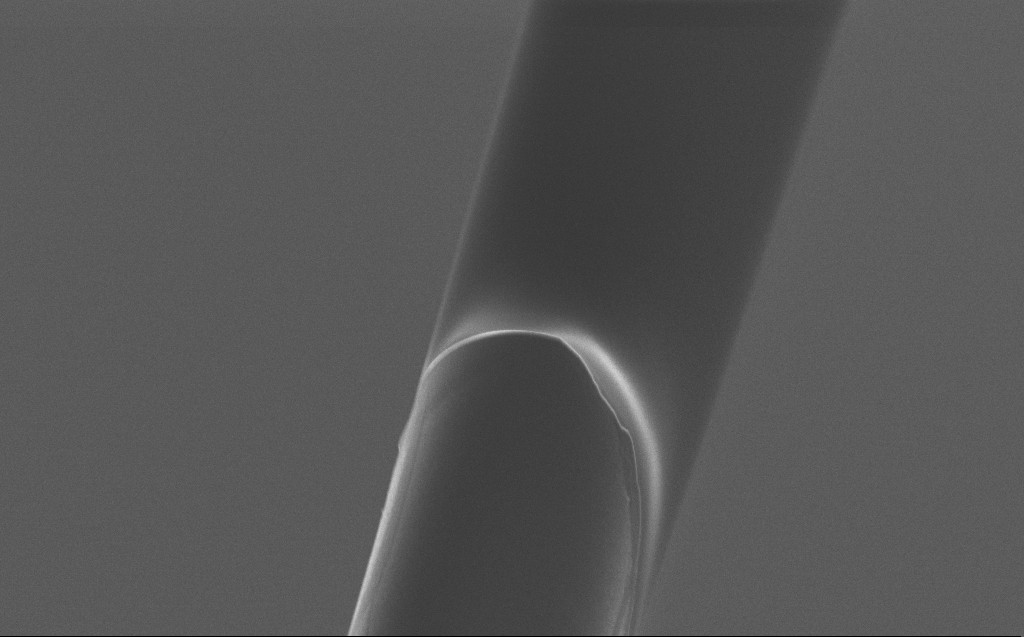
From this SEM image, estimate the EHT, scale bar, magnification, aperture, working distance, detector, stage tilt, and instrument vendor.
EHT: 5 kV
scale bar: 10000 nm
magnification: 4.3 K X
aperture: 30 µm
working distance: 6 mm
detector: InLens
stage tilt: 45°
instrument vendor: Zeiss